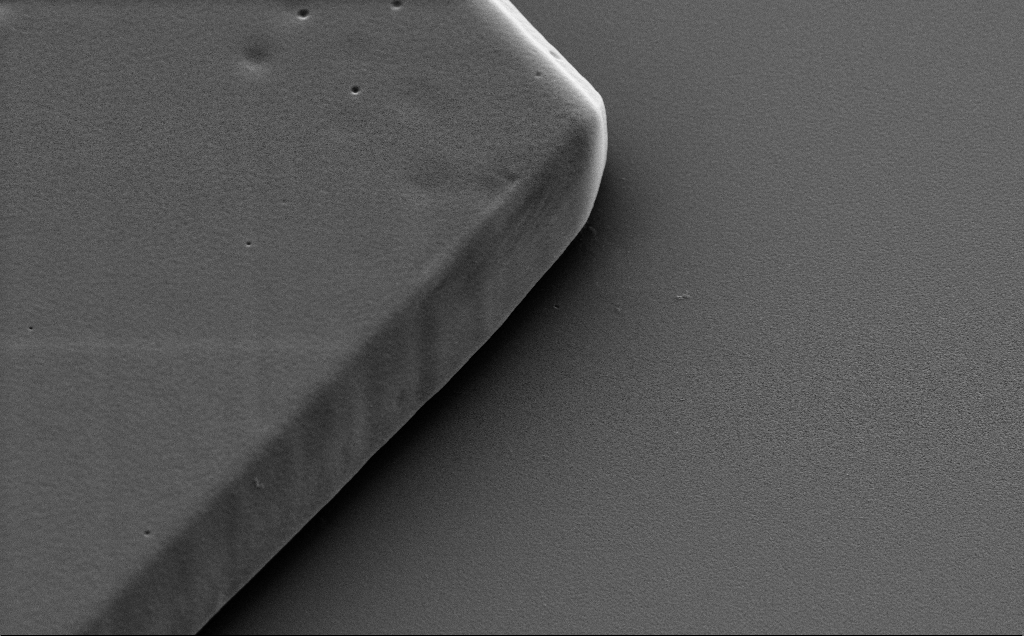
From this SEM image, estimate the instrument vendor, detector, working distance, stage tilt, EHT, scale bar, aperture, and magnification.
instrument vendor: Zeiss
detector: SE2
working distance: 9 mm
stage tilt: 40°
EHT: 5 kV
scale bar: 2000 nm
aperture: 30 µm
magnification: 10 K X